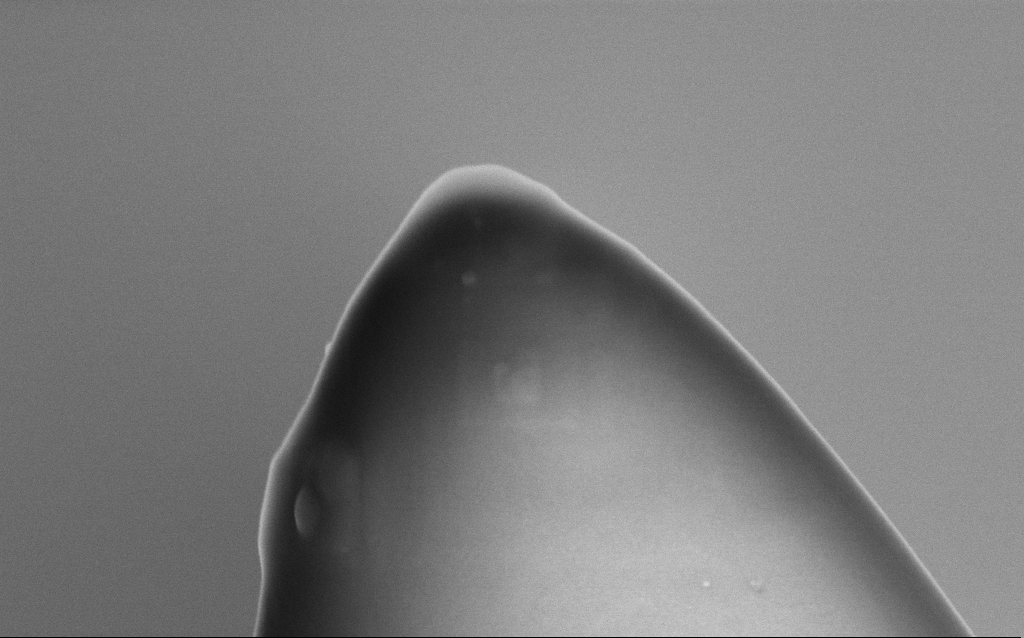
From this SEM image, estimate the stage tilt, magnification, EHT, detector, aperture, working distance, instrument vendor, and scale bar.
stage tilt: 0°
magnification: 149 K X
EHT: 5 kV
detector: InLens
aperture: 30 µm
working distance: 3.3 mm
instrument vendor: Zeiss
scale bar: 100 nm